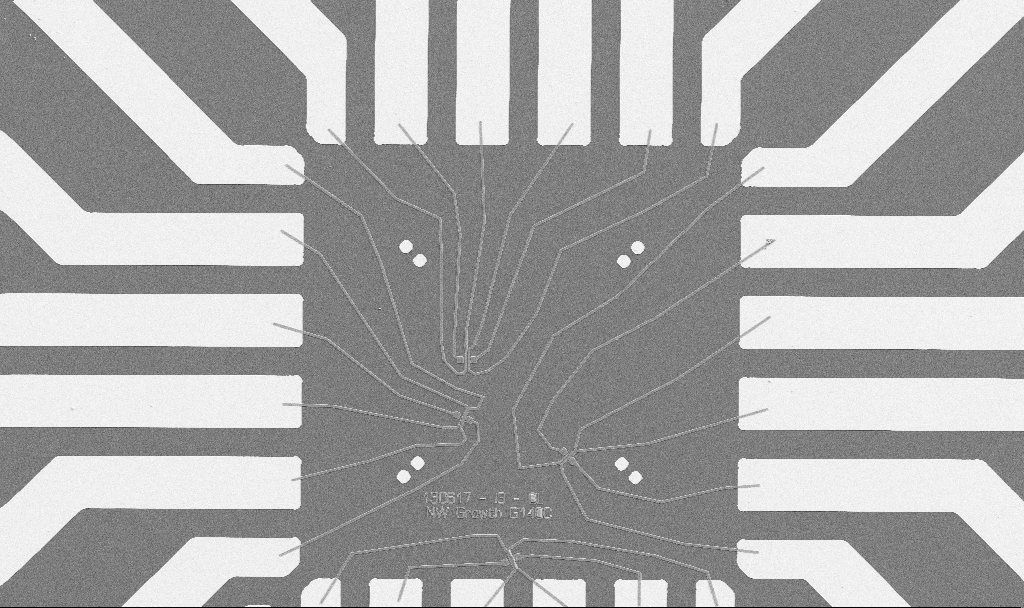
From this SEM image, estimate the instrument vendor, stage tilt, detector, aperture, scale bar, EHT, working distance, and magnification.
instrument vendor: Zeiss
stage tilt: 0°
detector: SE2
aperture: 30 µm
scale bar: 20000 nm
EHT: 5 kV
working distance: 10.7 mm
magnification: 1 K X